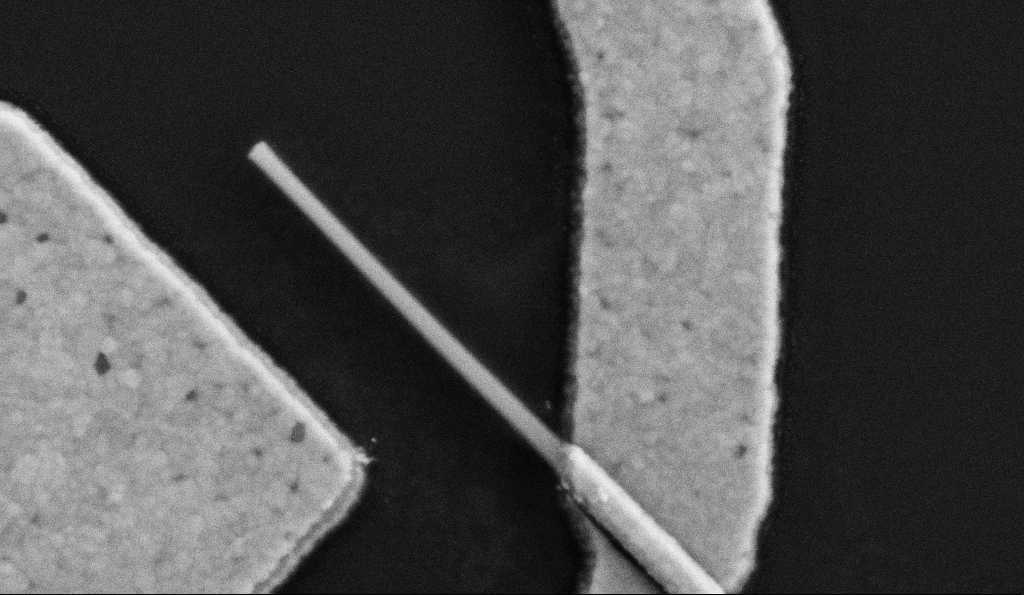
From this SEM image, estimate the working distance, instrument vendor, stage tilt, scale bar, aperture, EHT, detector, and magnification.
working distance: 9.6 mm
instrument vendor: Zeiss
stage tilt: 0°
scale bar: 200 nm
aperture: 30 µm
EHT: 5 kV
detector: SE2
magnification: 100 K X